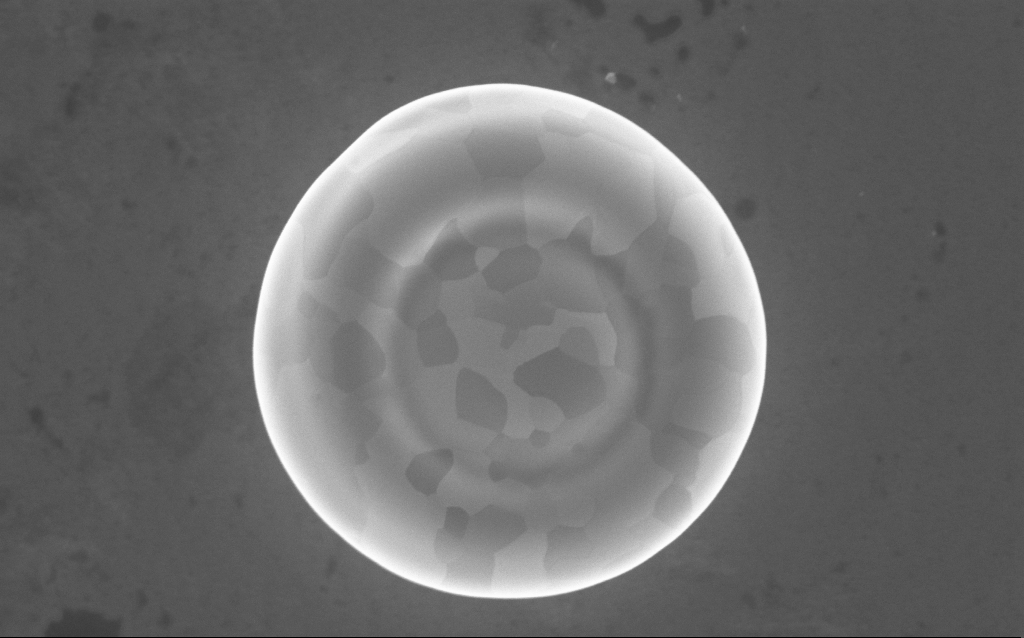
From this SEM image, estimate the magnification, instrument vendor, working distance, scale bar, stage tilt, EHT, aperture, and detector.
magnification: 50 K X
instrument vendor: Zeiss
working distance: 5 mm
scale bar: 1000 nm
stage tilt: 0°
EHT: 5 kV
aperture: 30 µm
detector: InLens